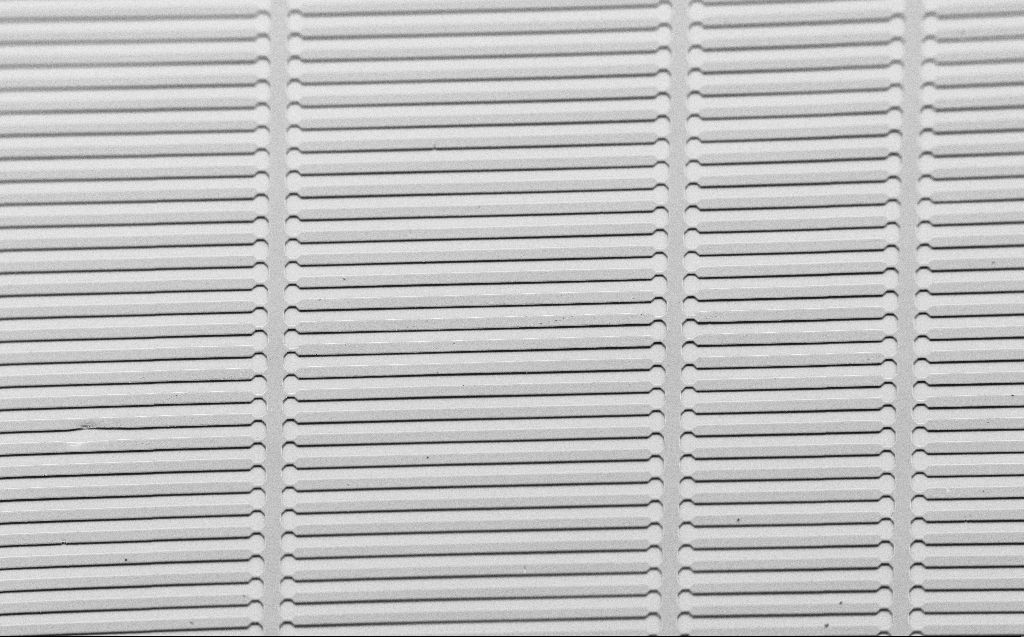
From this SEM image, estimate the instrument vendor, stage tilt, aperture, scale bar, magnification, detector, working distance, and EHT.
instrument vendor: Zeiss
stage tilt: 45°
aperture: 30 µm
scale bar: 100000 nm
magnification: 0.332 K X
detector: SE2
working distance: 6 mm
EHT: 1.7 kV